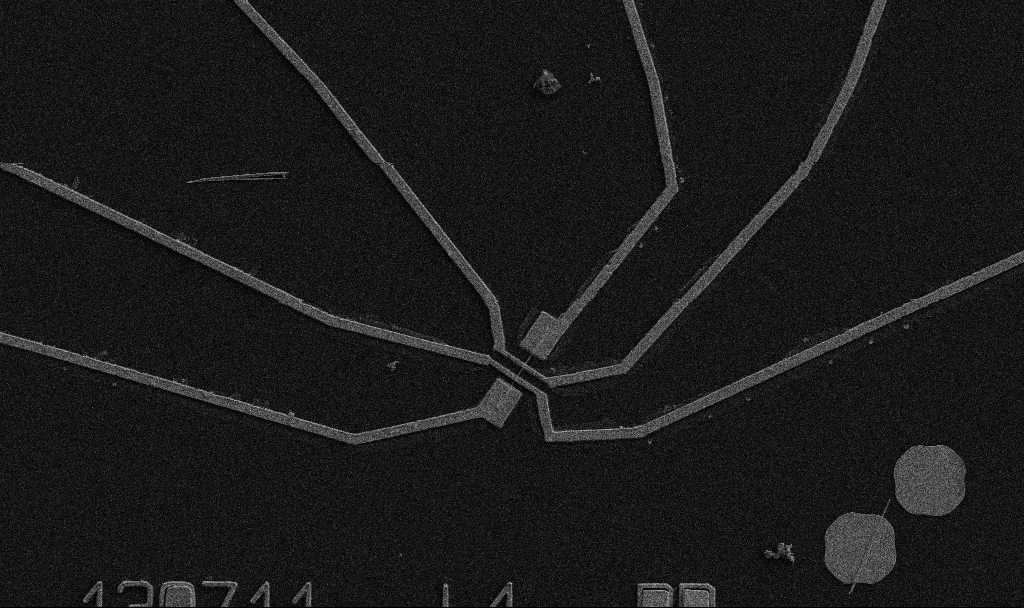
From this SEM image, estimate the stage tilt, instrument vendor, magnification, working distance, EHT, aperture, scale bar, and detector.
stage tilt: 0°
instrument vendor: Zeiss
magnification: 5 K X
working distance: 10.7 mm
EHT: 5 kV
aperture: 30 µm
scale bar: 10000 nm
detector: SE2